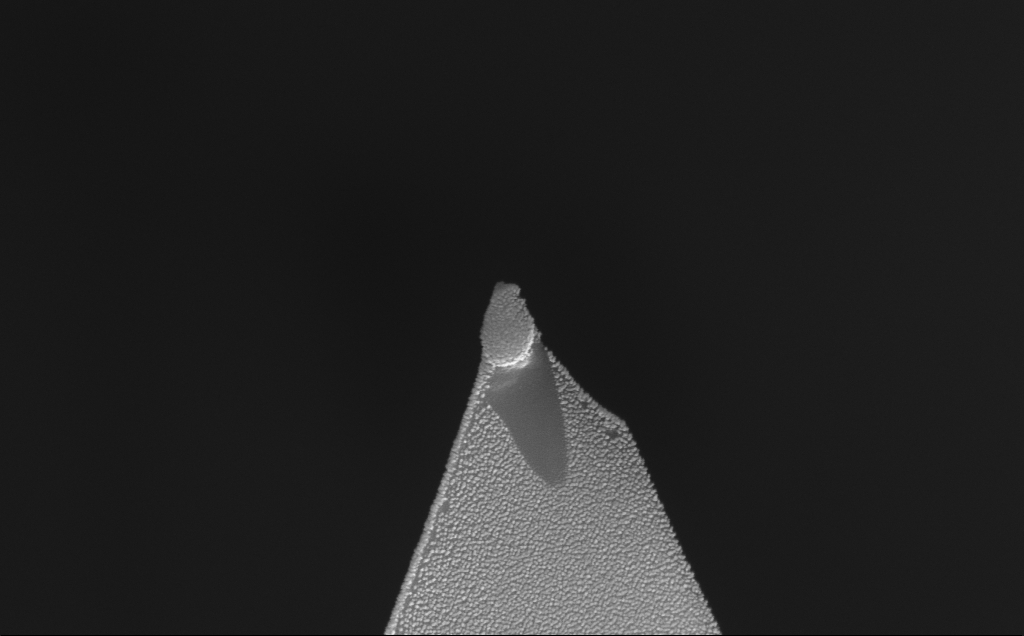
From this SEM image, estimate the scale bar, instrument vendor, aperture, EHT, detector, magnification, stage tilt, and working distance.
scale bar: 100 nm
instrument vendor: Zeiss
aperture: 30 µm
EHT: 10 kV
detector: InLens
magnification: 124.81 K X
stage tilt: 52.7°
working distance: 8 mm